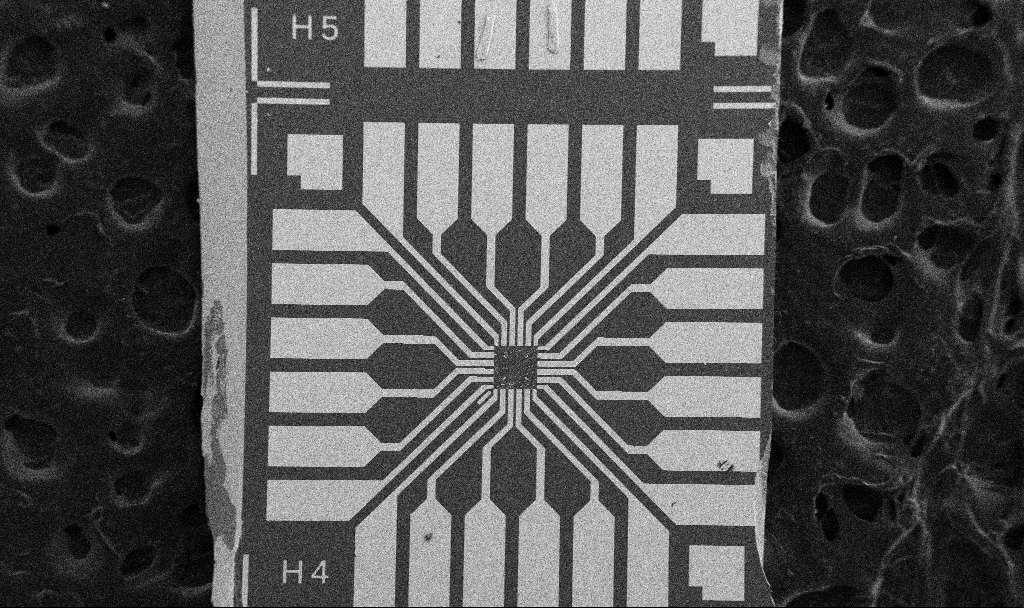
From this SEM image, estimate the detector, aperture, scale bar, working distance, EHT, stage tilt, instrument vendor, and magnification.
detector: SE2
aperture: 30 µm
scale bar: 200000 nm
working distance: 10.7 mm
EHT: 5 kV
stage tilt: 0°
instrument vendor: Zeiss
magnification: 0.1 K X